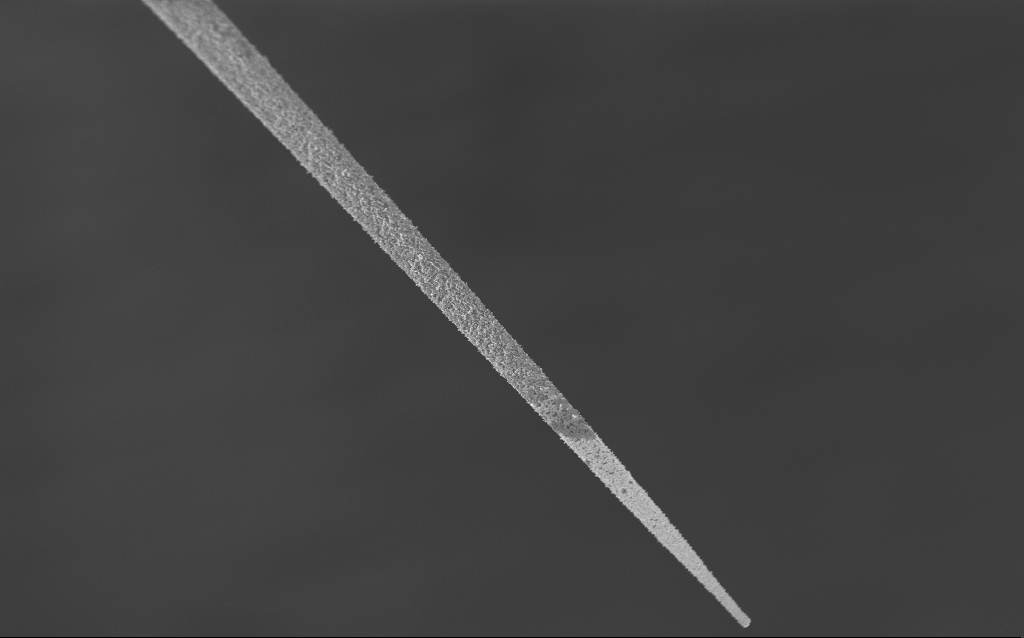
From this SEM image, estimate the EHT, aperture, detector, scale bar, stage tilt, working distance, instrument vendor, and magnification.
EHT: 1.5 kV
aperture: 30 µm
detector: InLens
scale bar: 100000 nm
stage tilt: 45°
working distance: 7.9 mm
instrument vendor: Zeiss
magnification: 0.5 K X